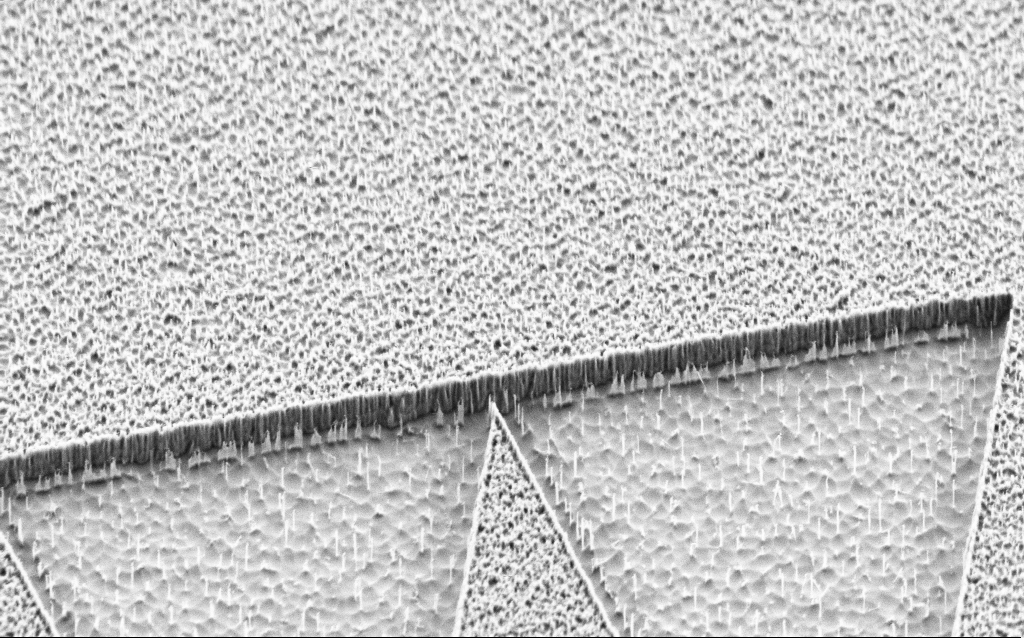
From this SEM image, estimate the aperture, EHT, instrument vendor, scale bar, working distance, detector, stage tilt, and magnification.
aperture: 30 µm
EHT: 2 kV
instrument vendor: Zeiss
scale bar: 1000 nm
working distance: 7 mm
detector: SE2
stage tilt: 45°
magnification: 19.04 K X